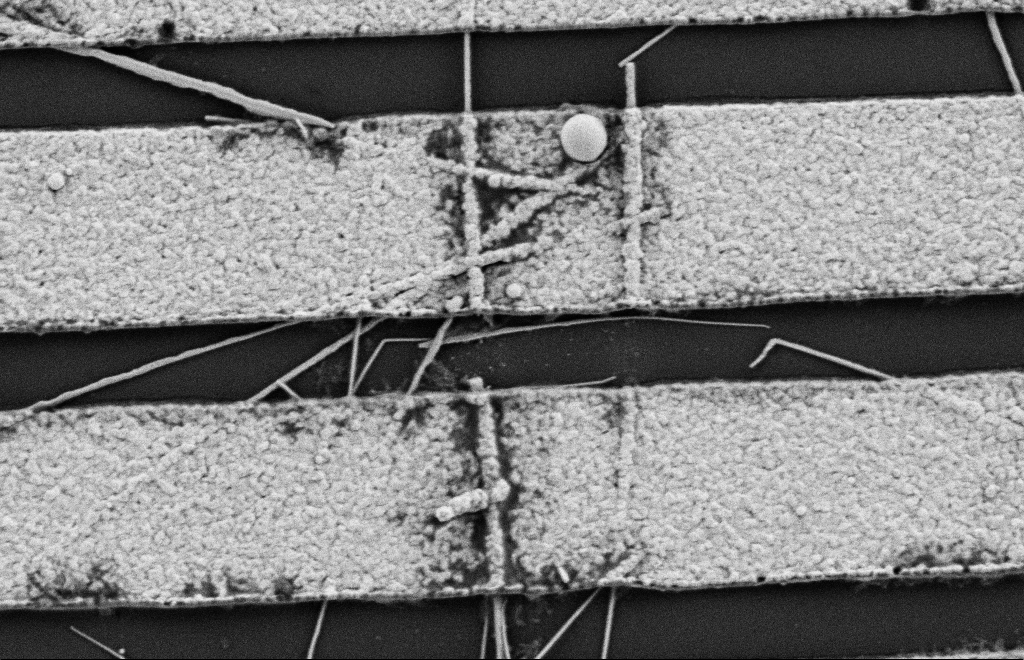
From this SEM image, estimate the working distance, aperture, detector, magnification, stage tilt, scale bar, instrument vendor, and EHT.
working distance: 9 mm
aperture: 20 µm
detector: SE2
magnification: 25.9 K X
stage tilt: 0°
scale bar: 1000 nm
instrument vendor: Zeiss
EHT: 2 kV